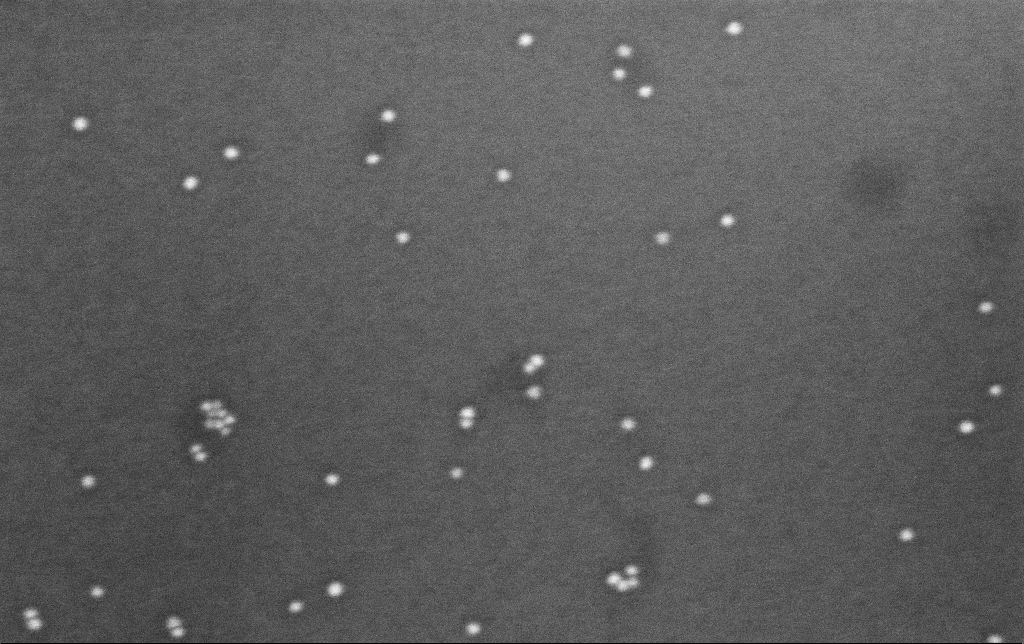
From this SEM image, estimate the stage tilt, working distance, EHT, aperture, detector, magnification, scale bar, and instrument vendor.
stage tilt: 0°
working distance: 6.6 mm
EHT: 8 kV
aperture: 30 µm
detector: InLens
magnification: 239.5 K X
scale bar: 200 nm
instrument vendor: Zeiss